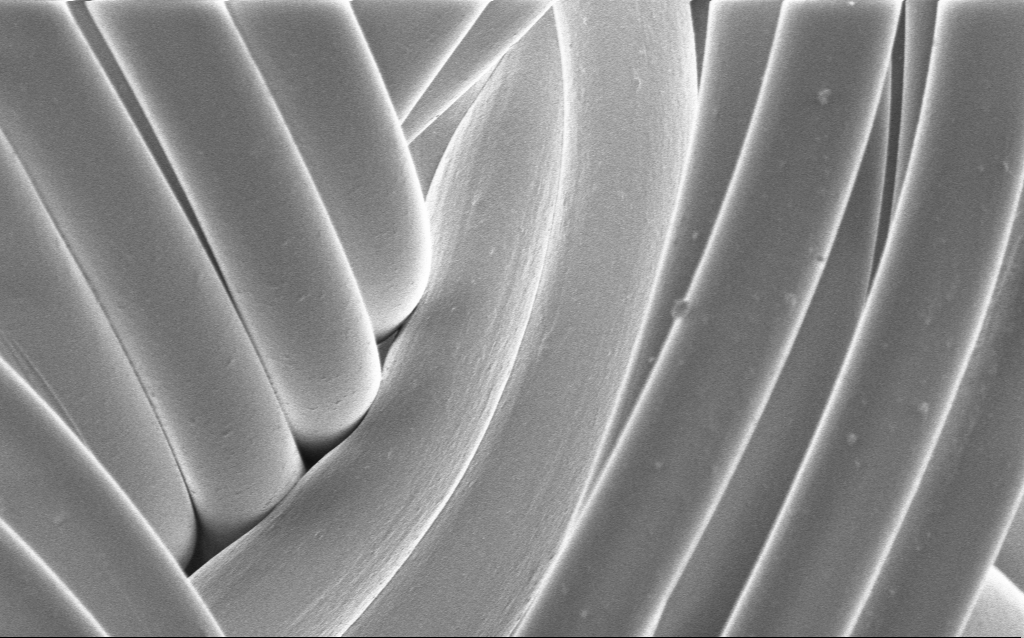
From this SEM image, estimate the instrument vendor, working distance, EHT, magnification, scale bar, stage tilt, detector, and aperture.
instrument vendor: Zeiss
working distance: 4 mm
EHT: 1 kV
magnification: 1.83 K X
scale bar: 20000 nm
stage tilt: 0°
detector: InLens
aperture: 30 µm